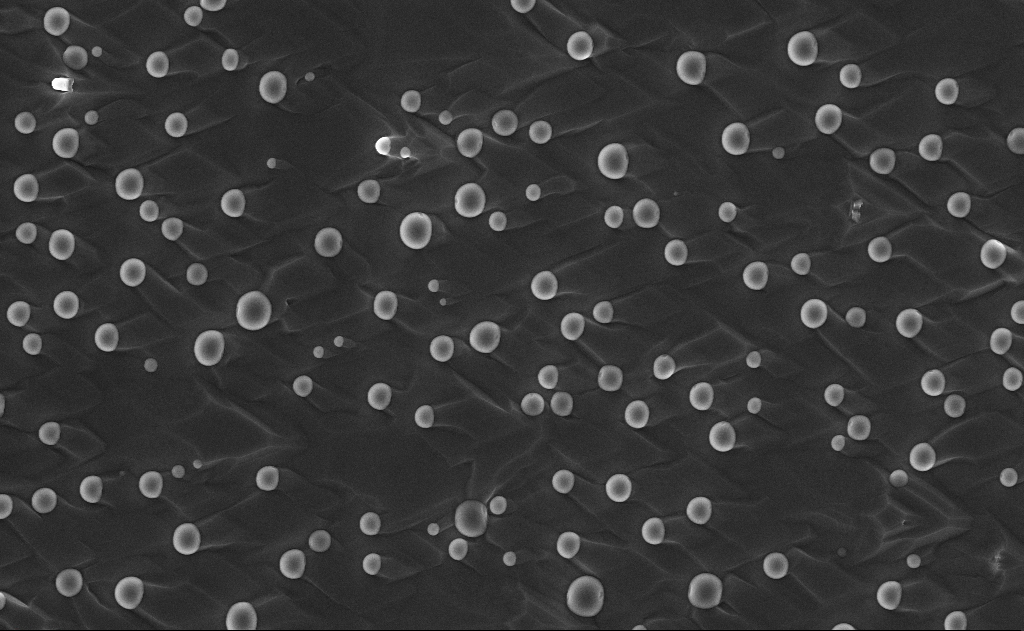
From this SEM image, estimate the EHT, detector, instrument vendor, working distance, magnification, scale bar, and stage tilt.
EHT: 10 kV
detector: InLens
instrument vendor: Zeiss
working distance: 10 mm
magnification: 10 K X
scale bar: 2000 nm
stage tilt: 0°